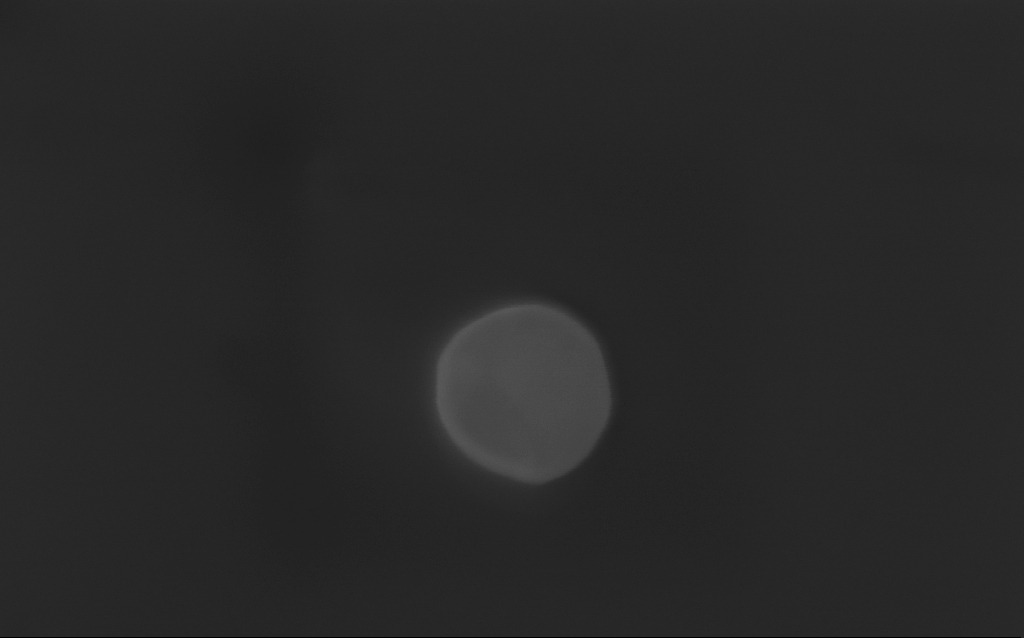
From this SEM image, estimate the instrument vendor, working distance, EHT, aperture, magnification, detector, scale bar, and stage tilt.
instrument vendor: Zeiss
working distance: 4 mm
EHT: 3 kV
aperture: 30 µm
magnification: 183.45 K X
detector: InLens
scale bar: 200 nm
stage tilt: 0°